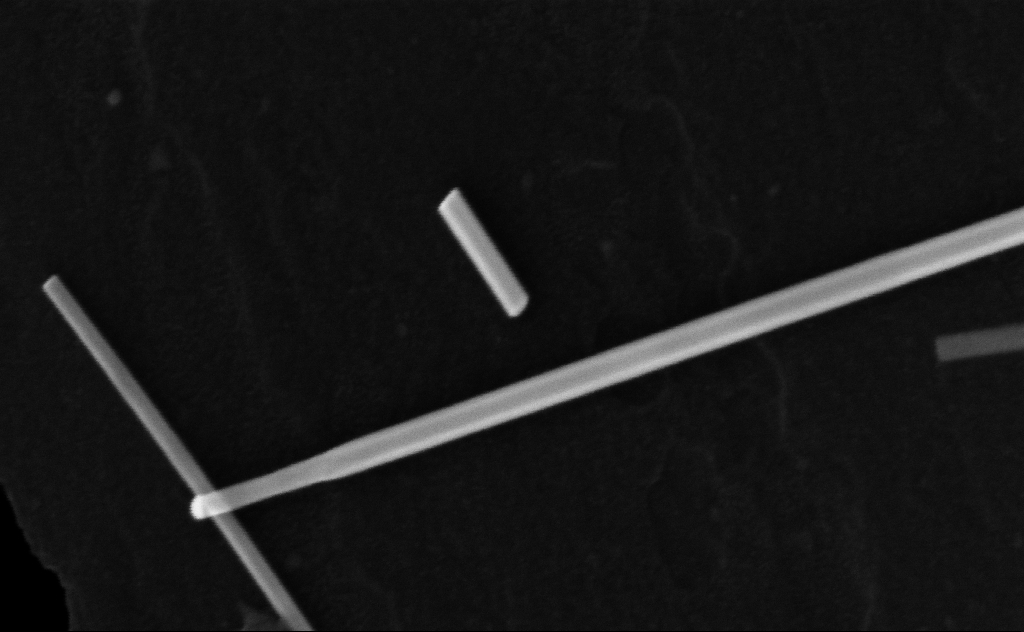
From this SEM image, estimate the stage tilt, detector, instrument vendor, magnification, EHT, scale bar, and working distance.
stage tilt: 0°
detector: InLens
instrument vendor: Zeiss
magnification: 275.05 K X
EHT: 10 kV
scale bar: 100 nm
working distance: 7 mm